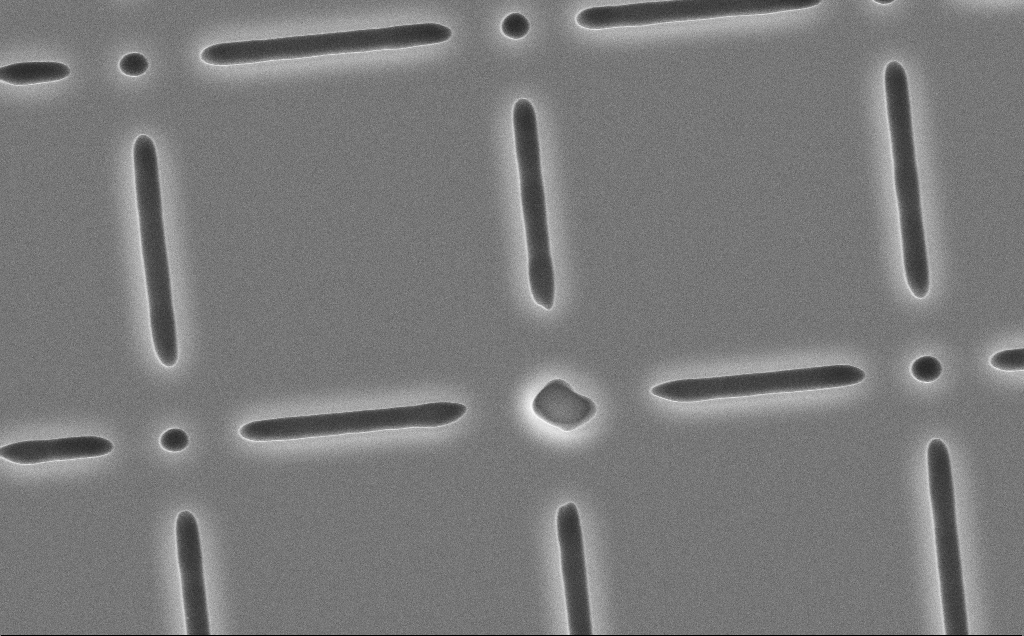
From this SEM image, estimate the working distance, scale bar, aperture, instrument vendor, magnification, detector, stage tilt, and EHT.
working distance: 15 mm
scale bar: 10000 nm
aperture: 30 µm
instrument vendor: Zeiss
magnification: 6.83 K X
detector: SE2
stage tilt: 0°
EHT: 10 kV